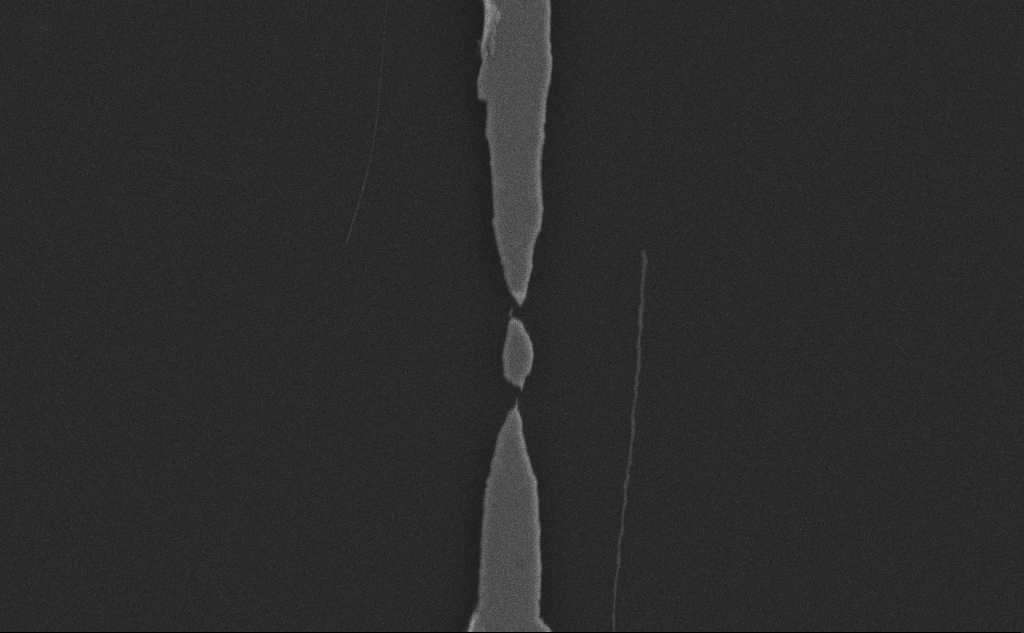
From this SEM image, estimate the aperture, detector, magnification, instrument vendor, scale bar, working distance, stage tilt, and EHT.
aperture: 30 µm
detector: SE2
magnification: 17.51 K X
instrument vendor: Zeiss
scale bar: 2000 nm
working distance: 6 mm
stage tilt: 0°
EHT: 10 kV